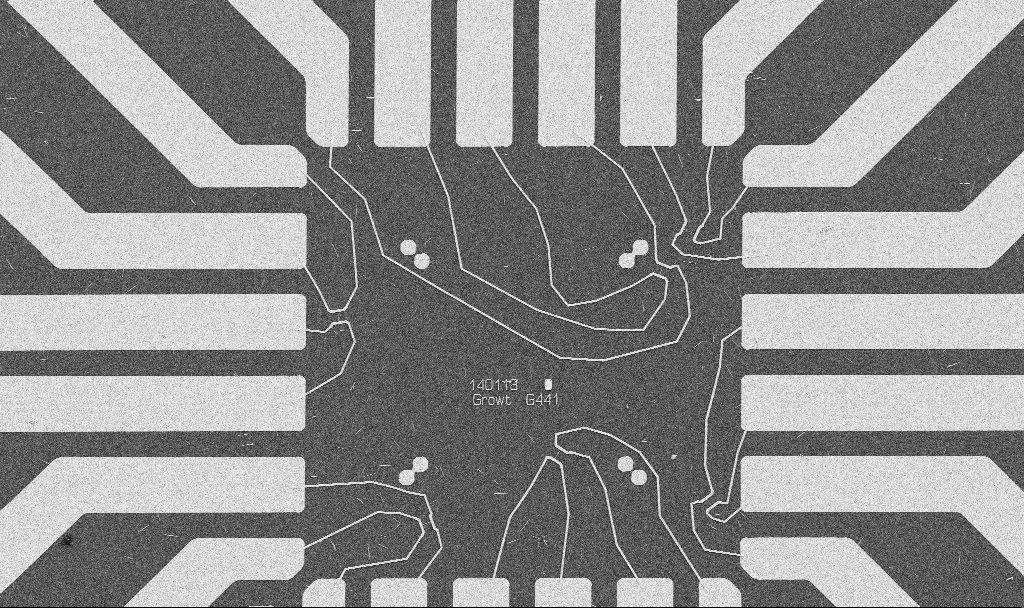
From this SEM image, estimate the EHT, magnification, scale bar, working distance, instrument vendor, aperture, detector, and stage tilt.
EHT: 5 kV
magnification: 1 K X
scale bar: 20000 nm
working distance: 10.7 mm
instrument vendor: Zeiss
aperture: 30 µm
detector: SE2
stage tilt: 0°